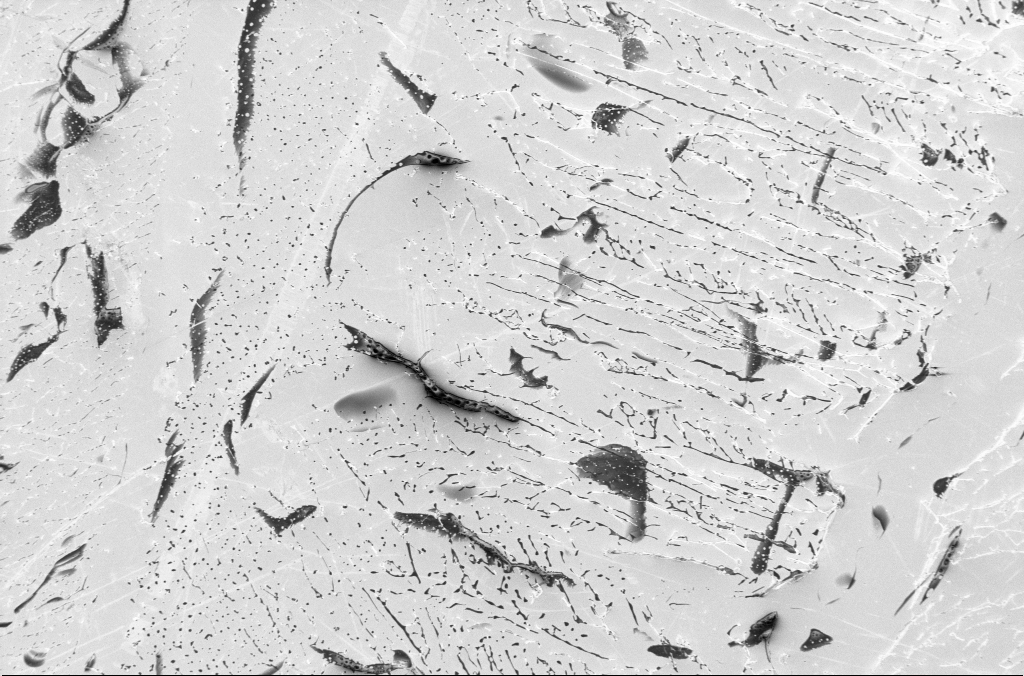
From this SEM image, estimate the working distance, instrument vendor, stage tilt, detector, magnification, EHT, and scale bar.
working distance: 4.5 mm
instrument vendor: Zeiss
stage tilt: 0°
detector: InLens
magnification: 1 K X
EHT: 1.8 kV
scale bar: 20000 nm